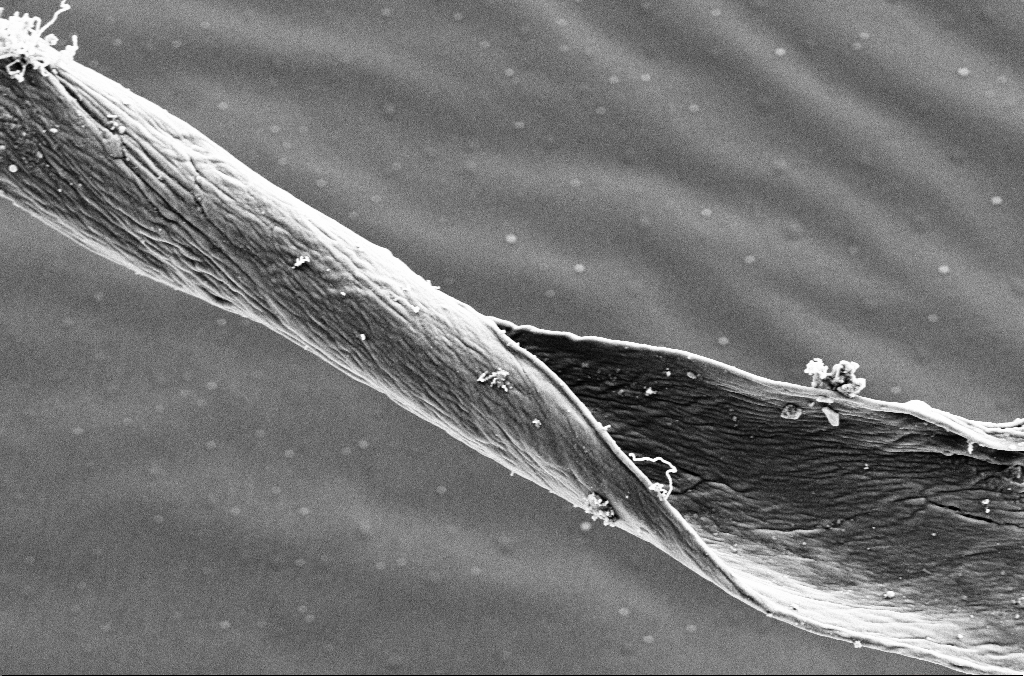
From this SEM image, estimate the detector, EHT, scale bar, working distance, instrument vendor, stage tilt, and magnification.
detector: SE2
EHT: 3 kV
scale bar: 2000 nm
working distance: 5 mm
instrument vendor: Zeiss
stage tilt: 0°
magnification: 10 K X